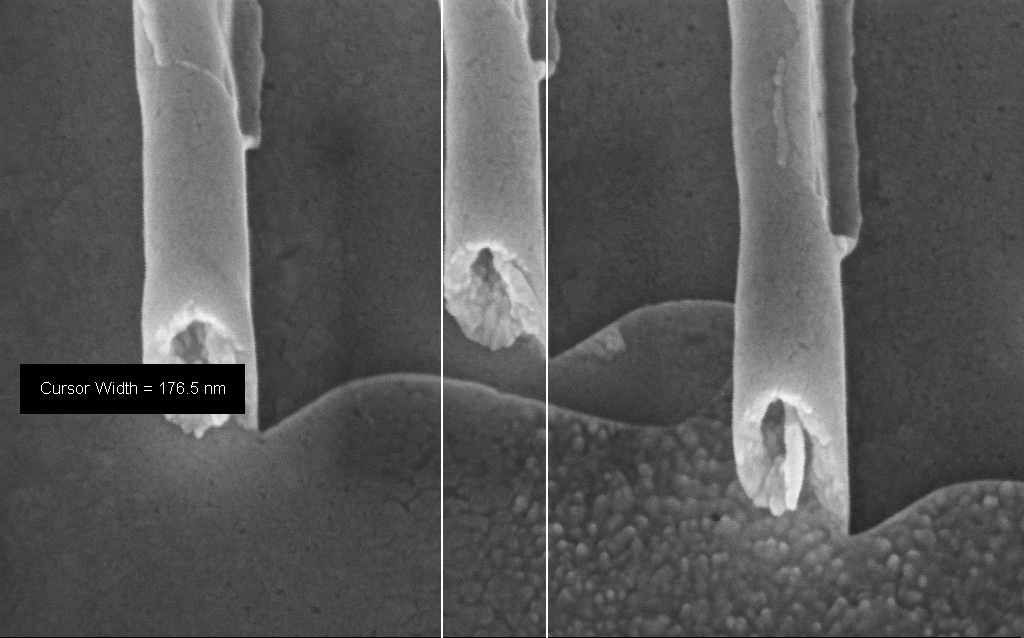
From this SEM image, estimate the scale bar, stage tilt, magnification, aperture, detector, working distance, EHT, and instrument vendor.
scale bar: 200 nm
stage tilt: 45°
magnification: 218.43 K X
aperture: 30 µm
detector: InLens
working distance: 7 mm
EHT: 10 kV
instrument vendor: Zeiss